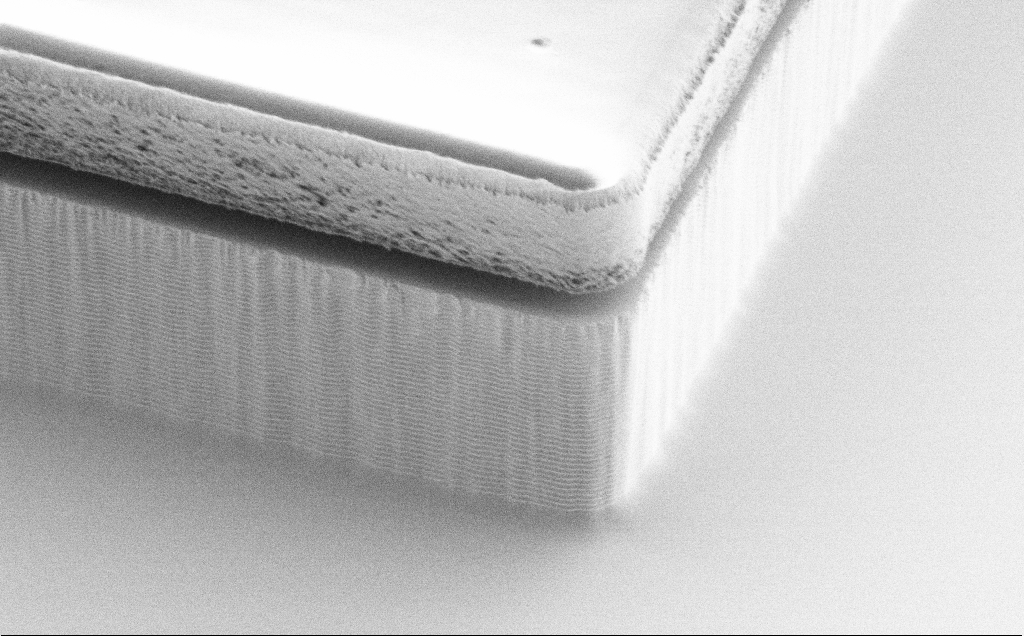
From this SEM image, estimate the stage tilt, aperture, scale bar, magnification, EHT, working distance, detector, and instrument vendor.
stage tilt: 45°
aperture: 30 µm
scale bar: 2000 nm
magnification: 7.44 K X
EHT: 1.2 kV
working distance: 8 mm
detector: SE2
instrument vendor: Zeiss